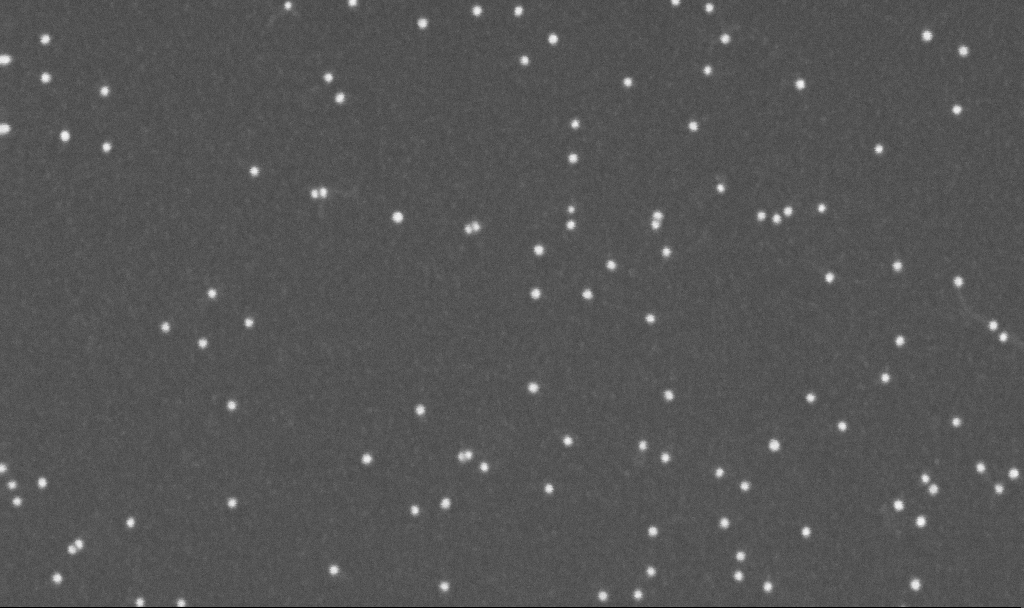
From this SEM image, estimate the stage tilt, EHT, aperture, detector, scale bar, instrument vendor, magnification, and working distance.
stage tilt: -0°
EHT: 10 kV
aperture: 30 µm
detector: InLens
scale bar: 200 nm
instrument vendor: Zeiss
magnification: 300 K X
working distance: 3.3 mm